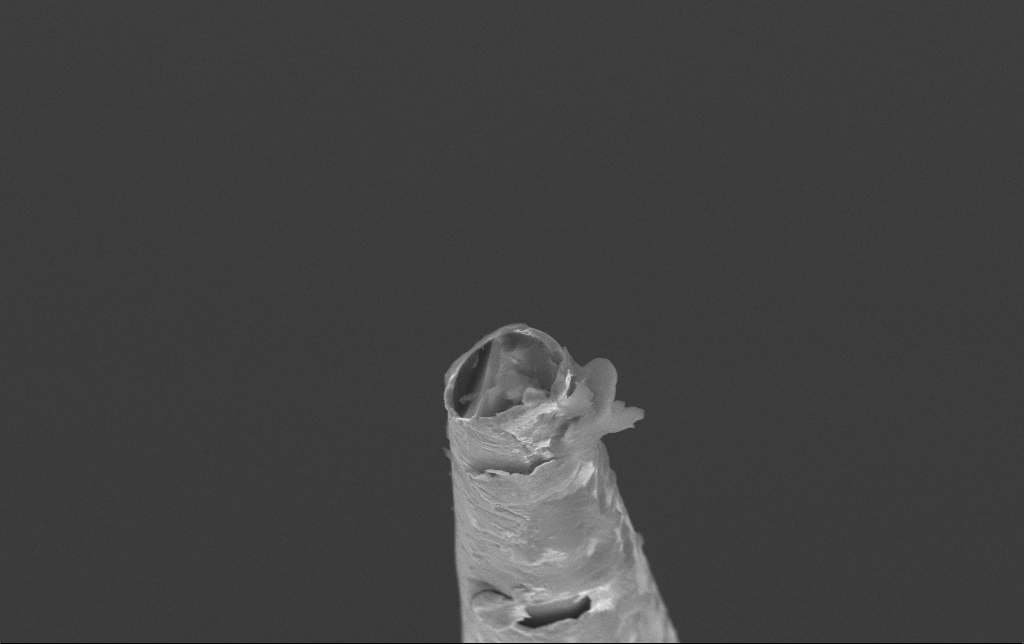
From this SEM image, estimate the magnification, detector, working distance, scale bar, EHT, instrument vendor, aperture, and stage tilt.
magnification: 10 K X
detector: SE2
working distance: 9.9 mm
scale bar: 2000 nm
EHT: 10 kV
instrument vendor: Zeiss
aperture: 30 µm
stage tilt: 69.3°